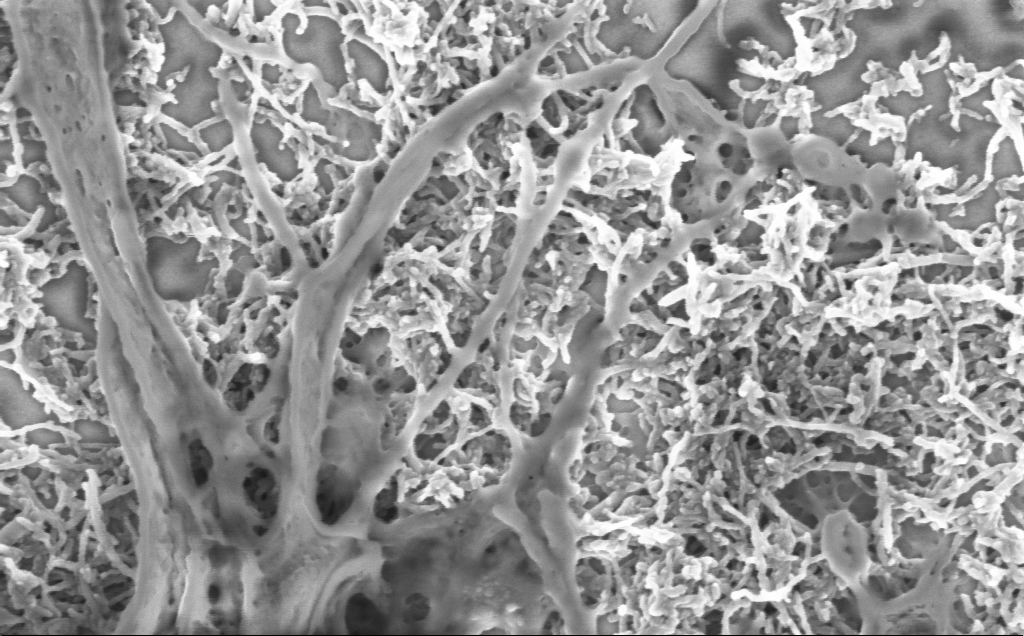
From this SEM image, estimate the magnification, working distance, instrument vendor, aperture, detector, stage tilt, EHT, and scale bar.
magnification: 75 K X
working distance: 7 mm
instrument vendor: Zeiss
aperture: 30 µm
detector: InLens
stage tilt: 0°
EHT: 2 kV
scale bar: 200 nm